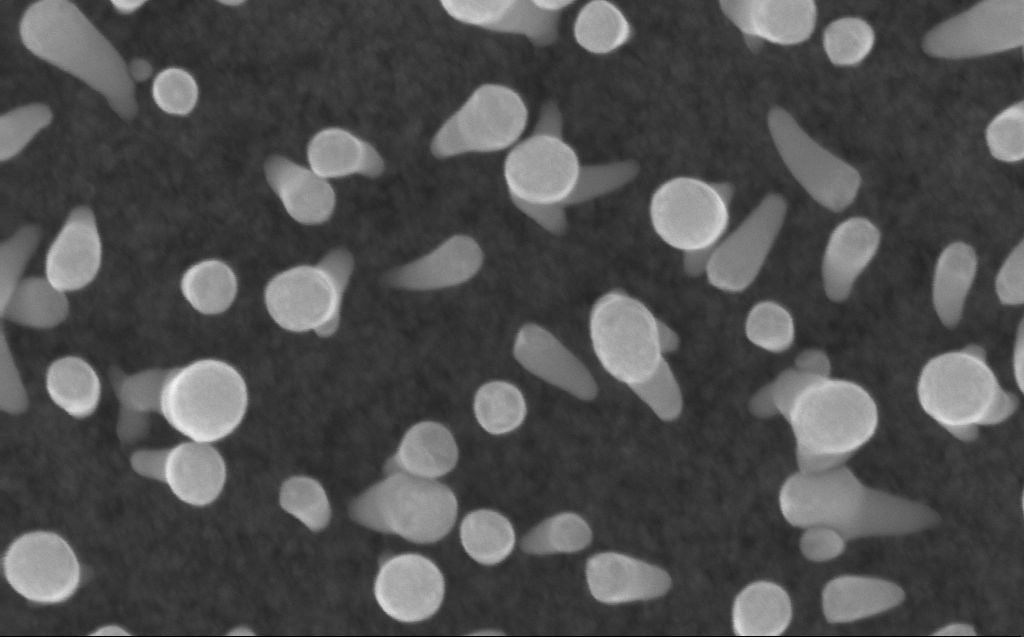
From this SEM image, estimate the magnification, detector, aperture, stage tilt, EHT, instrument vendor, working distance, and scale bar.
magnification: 200 K X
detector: InLens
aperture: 30 µm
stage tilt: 0°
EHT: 10 kV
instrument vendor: Zeiss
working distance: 3 mm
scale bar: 100 nm